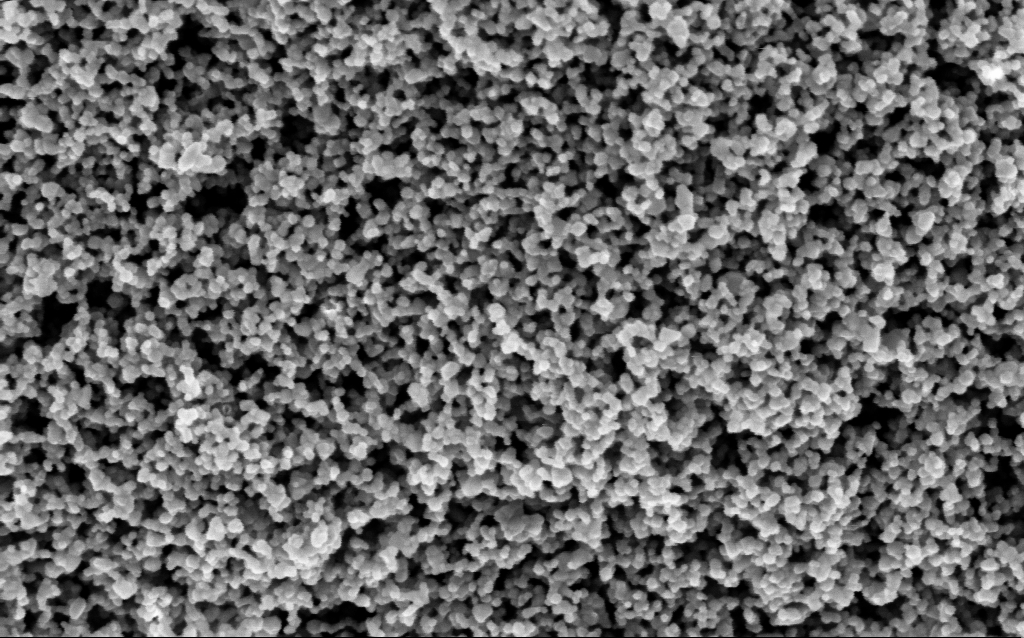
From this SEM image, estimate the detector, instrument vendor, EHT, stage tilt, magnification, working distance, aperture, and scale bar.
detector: InLens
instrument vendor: Zeiss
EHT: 3 kV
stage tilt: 0°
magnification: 130 K X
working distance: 7.5 mm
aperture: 30 µm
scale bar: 100 nm